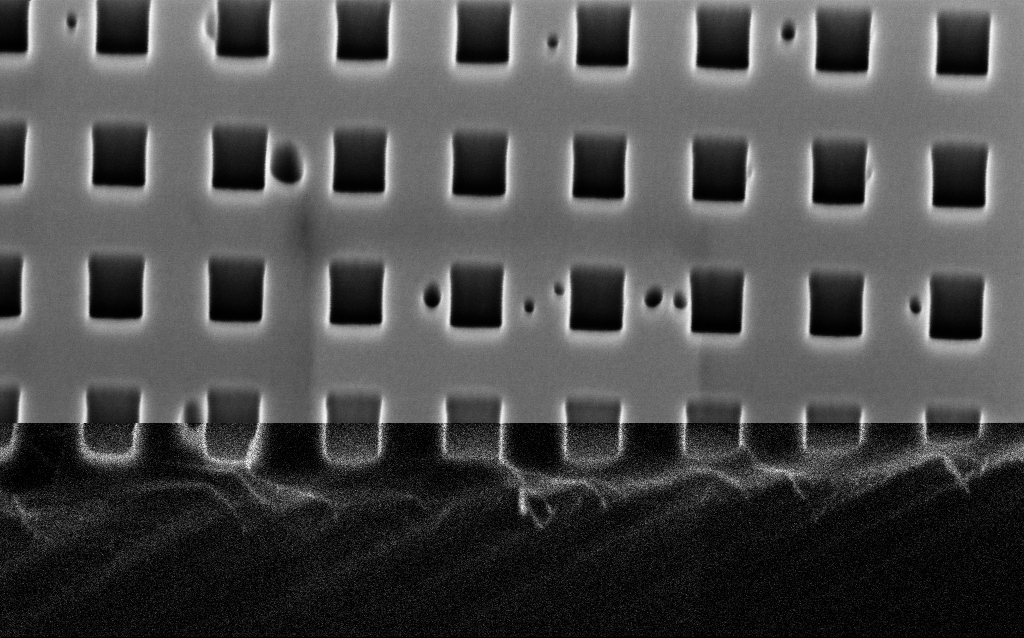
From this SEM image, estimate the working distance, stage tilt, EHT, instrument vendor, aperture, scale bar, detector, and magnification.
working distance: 3.6 mm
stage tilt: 45°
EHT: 2 kV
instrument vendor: Zeiss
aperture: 30 µm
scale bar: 200 nm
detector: InLens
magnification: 88.72 K X